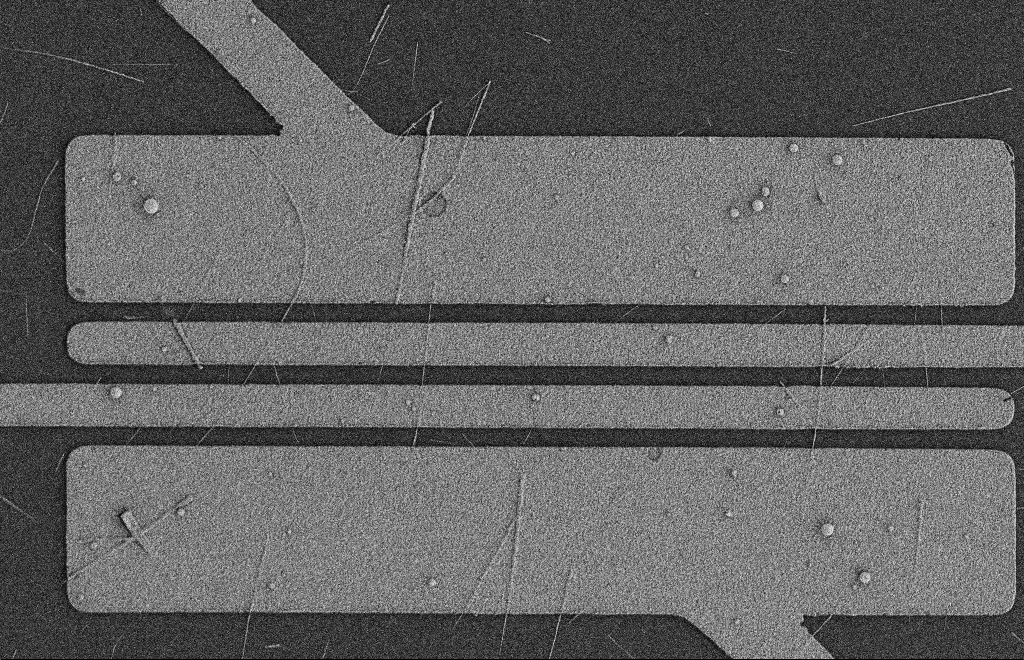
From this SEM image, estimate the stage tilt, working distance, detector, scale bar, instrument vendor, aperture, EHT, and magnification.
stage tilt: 0°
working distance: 9 mm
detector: SE2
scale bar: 2000 nm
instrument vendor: Zeiss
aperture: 20 µm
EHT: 2 kV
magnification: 5.69 K X